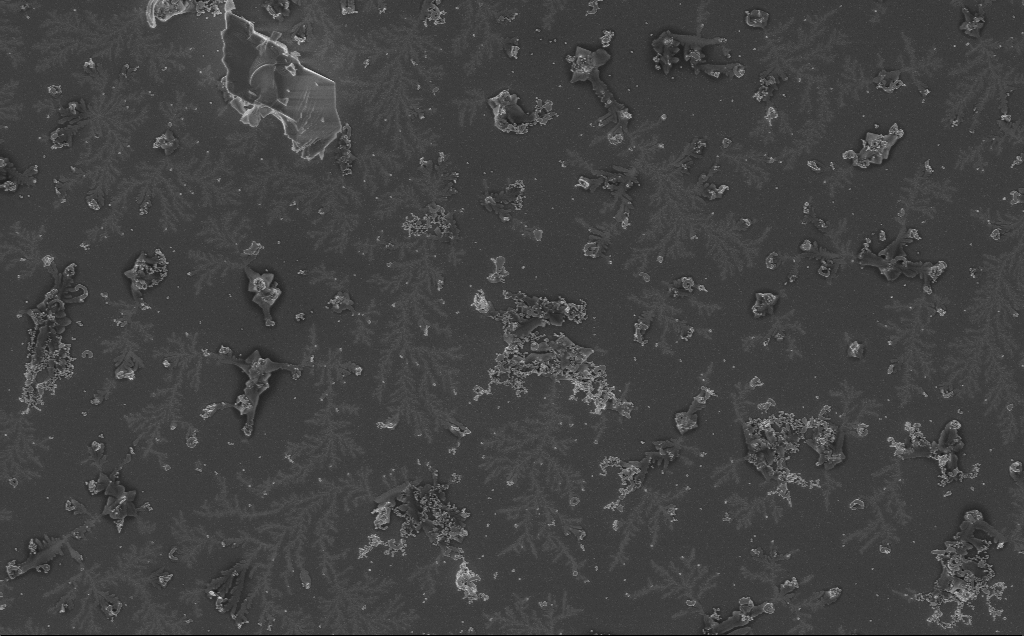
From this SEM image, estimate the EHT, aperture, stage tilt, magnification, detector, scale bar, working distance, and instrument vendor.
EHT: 5 kV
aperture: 30 µm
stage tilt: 0°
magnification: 5 K X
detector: InLens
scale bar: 10000 nm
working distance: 3 mm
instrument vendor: Zeiss